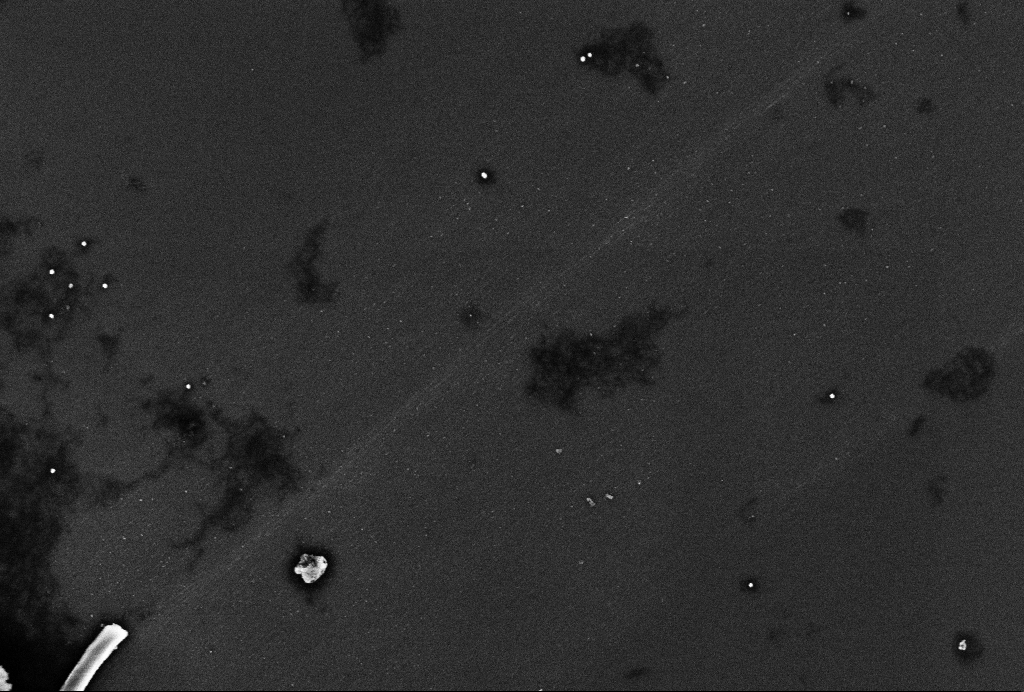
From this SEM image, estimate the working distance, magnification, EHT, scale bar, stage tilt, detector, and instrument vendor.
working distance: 3.3 mm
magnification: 62.51 K X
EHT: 2 kV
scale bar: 200 nm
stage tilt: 0°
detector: InLens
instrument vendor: Zeiss